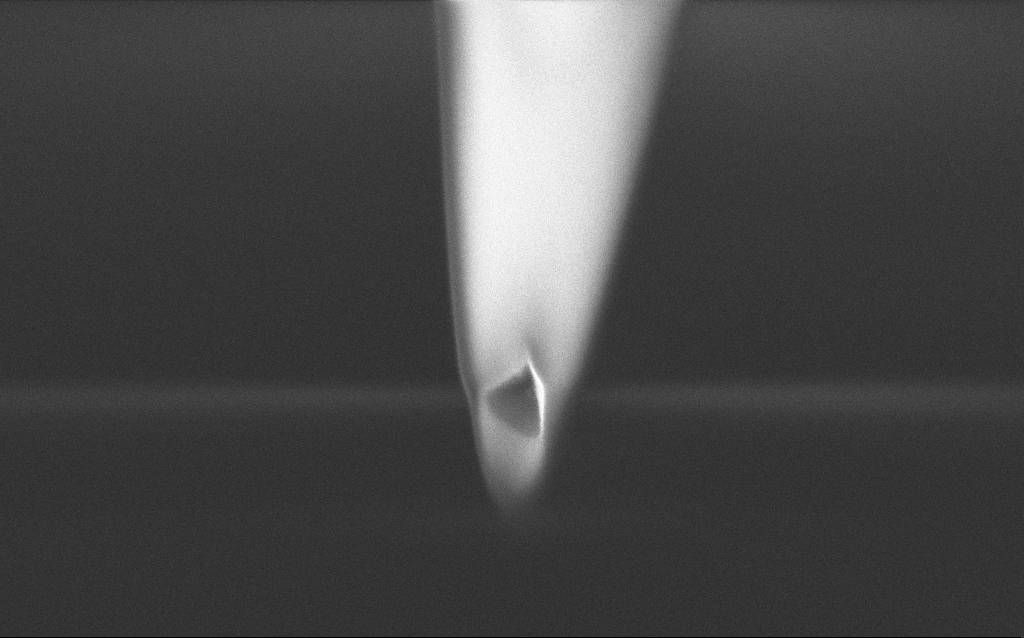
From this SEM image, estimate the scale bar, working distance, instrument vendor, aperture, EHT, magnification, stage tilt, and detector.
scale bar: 200 nm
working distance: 5 mm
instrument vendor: Zeiss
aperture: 30 µm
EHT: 1 kV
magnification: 104.96 K X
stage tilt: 45°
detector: InLens